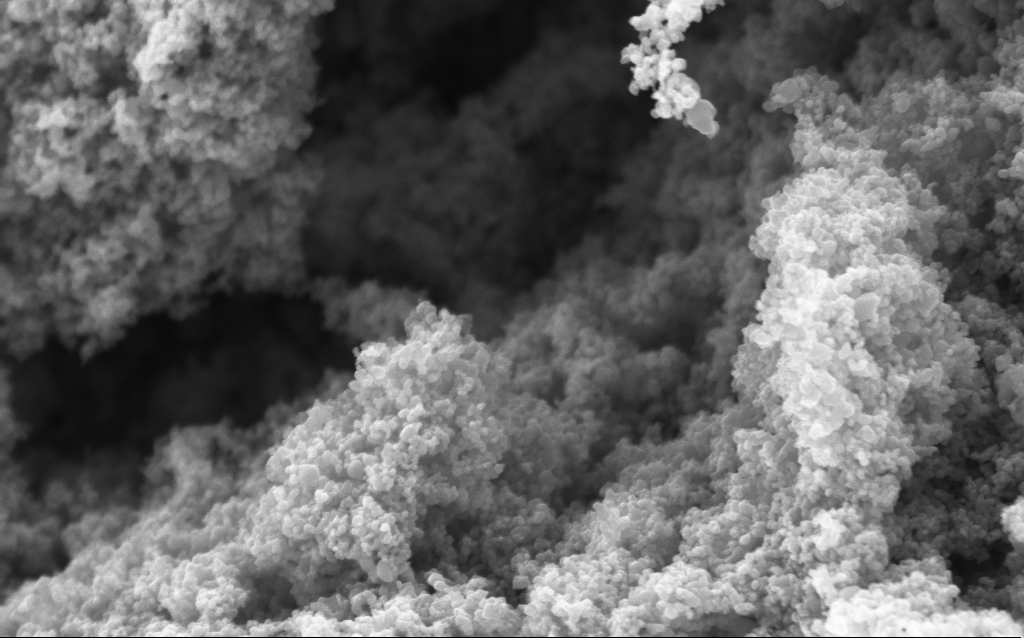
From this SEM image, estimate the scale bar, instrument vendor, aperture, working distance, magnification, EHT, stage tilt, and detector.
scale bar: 200 nm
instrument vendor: Zeiss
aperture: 30 µm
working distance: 4.4 mm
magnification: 114.68 K X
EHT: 5 kV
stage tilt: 0°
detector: InLens